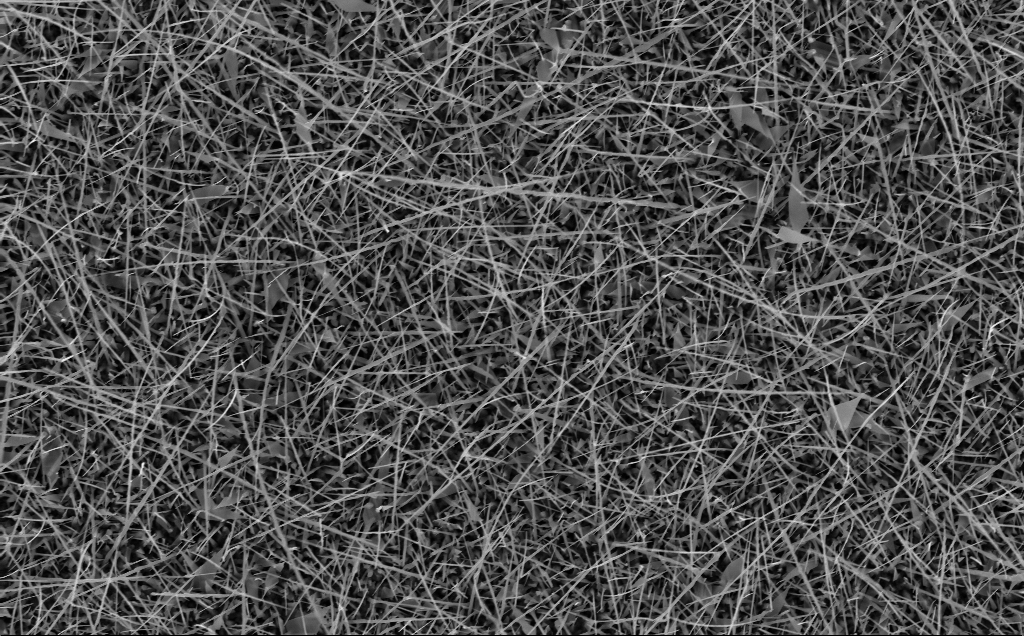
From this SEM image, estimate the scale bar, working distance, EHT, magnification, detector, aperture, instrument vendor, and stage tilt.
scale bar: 2000 nm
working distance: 6 mm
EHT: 10 kV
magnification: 10 K X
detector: InLens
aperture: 30 µm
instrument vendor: Zeiss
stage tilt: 0°